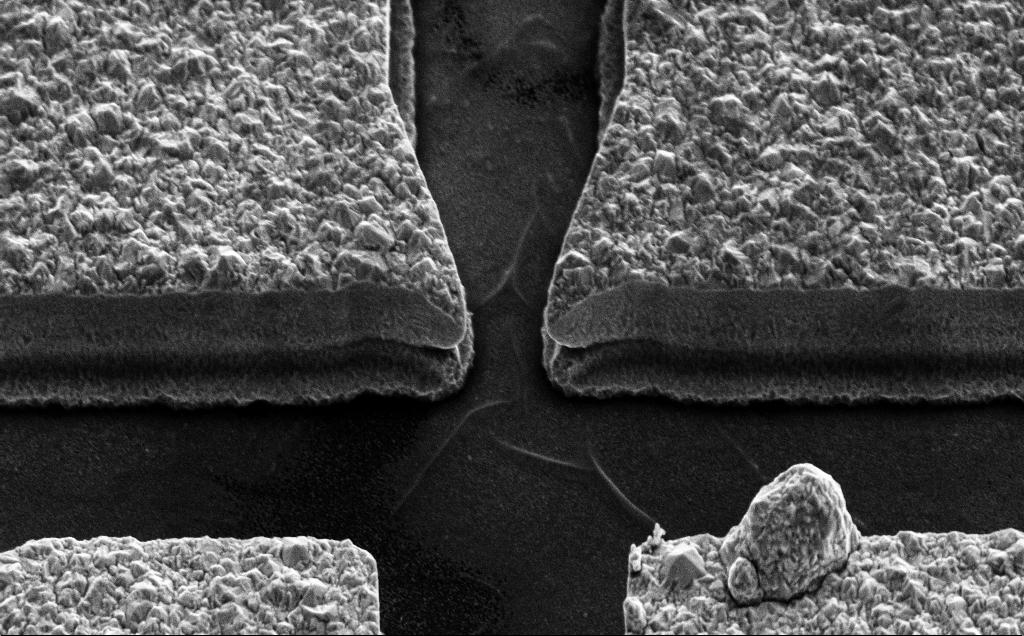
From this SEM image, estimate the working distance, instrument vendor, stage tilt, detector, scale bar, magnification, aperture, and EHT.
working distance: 15 mm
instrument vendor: Zeiss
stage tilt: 45°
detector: SE2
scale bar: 2000 nm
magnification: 9.13 K X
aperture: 30 µm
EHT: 10 kV